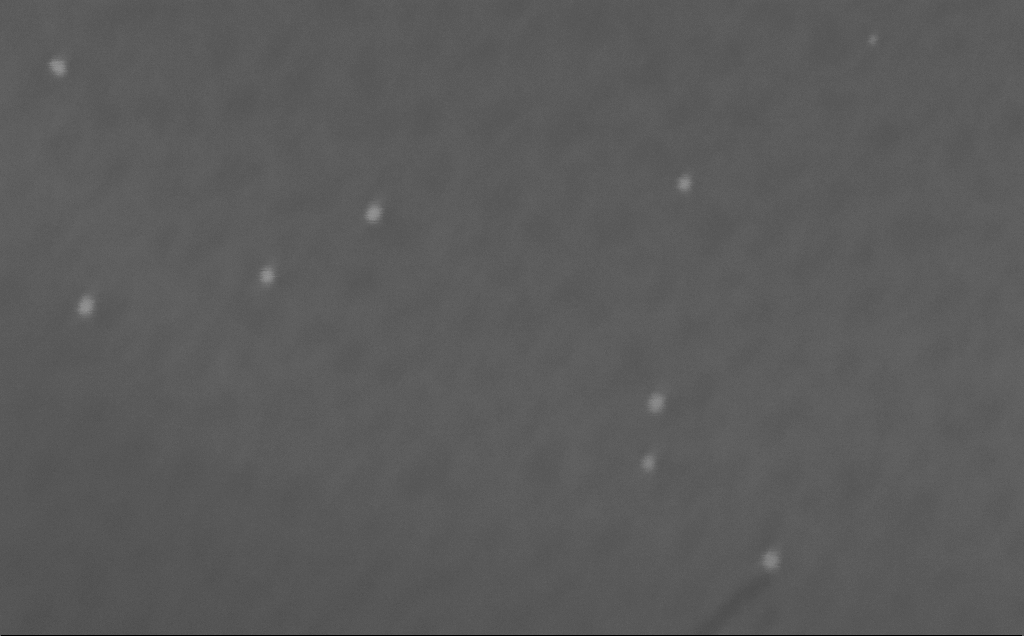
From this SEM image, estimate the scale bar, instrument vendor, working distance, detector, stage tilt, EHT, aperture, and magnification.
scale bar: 20 nm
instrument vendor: Zeiss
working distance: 6 mm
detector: InLens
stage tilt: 0°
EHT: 10 kV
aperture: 30 µm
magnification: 731.21 K X